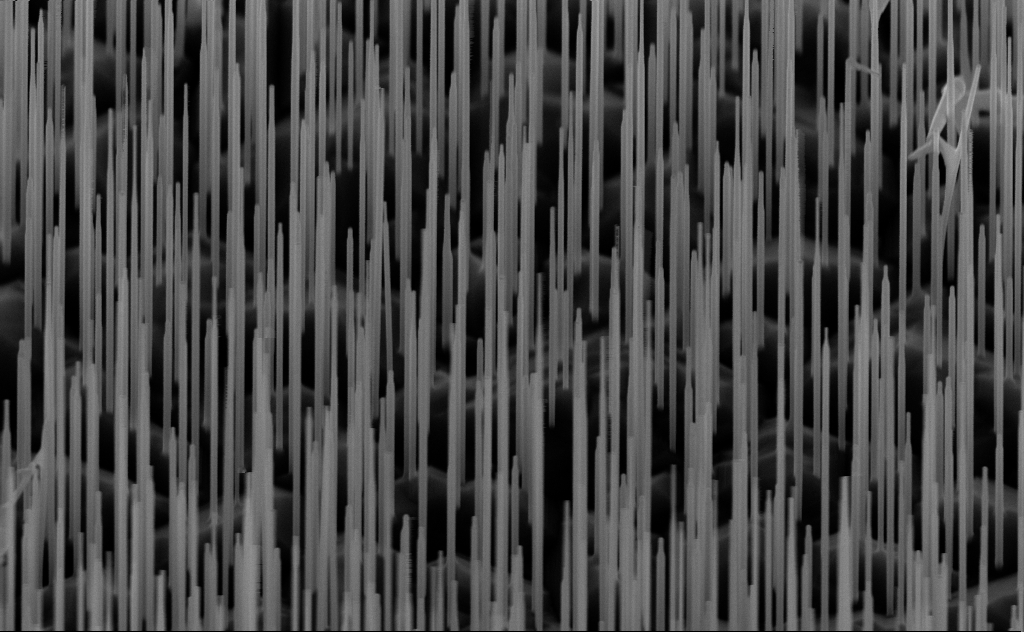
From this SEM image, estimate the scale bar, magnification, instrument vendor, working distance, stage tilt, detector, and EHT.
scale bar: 1000 nm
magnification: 40 K X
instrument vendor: Zeiss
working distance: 6 mm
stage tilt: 44.2°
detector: InLens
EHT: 10 kV